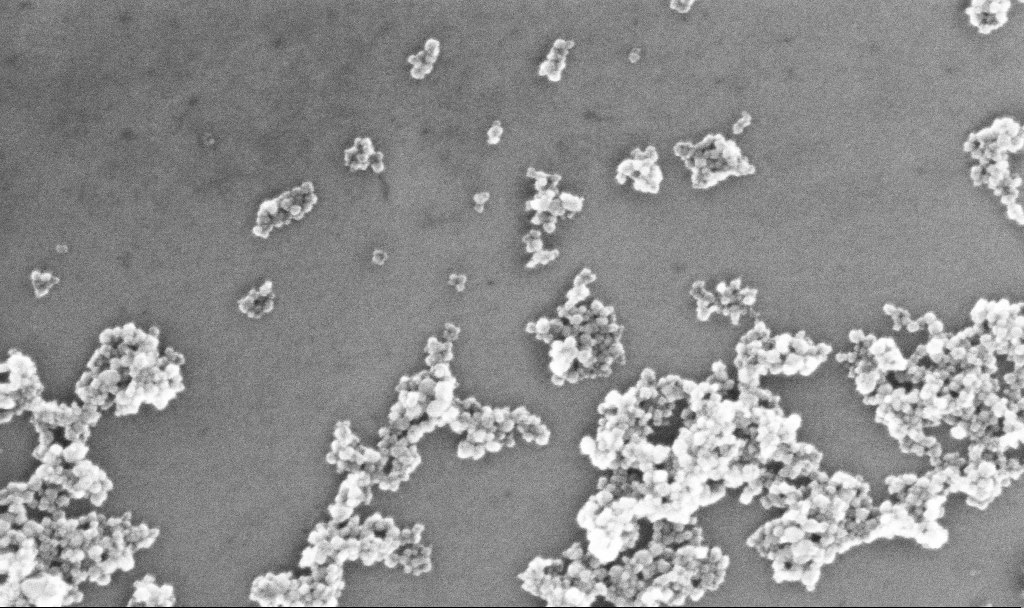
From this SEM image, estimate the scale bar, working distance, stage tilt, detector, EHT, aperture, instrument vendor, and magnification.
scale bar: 200 nm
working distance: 5.2 mm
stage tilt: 0°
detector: InLens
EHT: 10 kV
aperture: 30 µm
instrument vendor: Zeiss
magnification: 209.17 K X